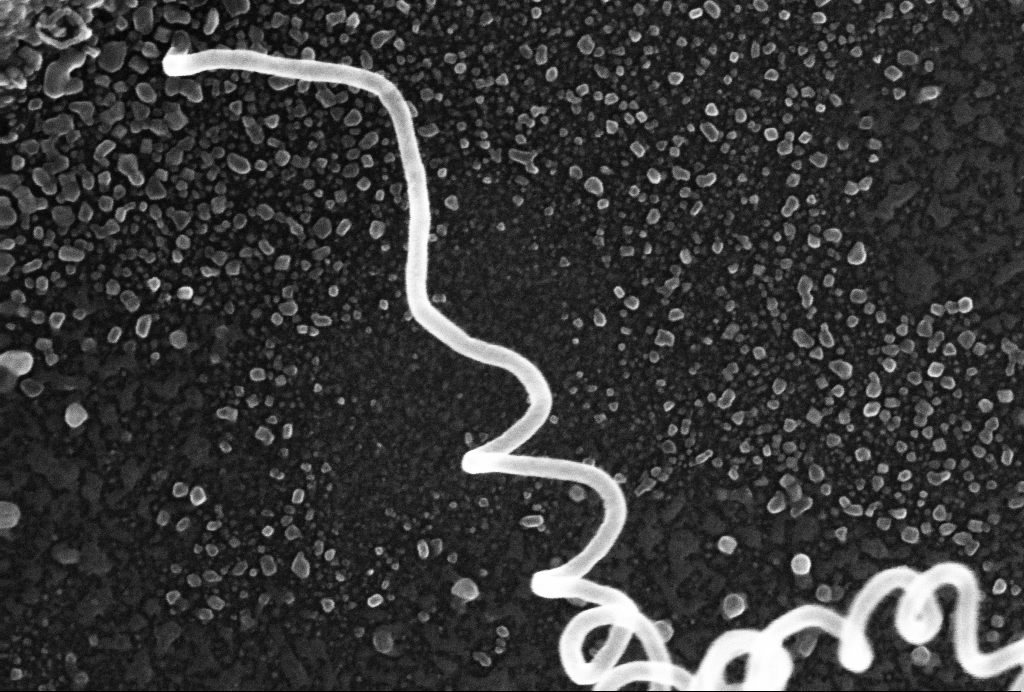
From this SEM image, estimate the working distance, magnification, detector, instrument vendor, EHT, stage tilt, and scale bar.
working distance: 3 mm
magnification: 190.67 K X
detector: InLens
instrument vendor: Zeiss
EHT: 10 kV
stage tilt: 0°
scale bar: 300 nm